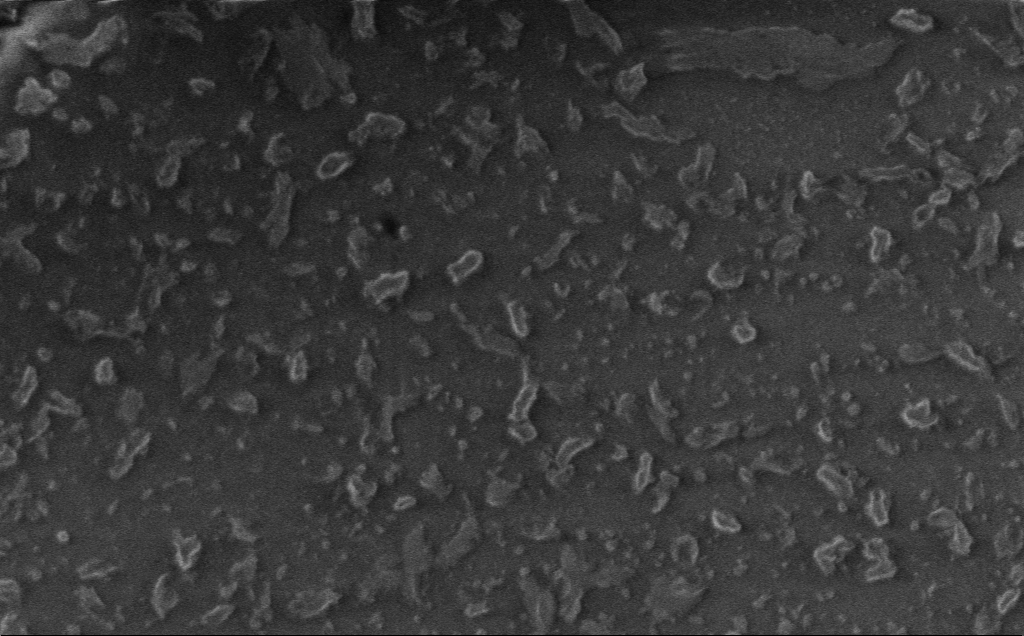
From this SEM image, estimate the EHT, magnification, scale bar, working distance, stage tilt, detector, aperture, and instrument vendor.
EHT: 1 kV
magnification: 12.52 K X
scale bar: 2000 nm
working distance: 3 mm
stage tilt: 0°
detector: InLens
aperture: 30 µm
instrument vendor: Zeiss